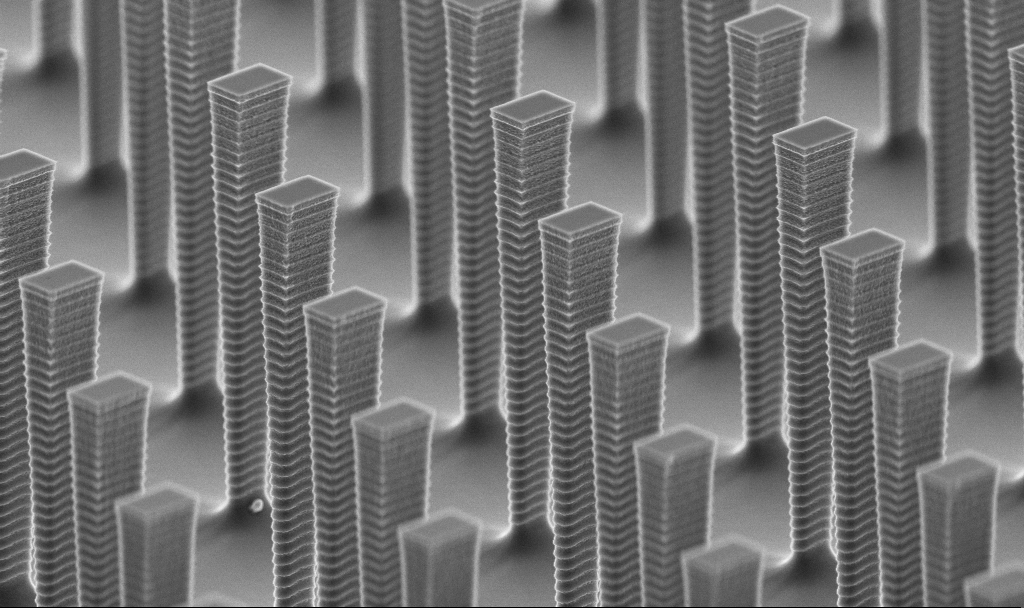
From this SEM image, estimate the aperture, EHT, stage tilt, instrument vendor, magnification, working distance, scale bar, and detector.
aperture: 30 µm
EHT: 5 kV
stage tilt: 70°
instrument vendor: Zeiss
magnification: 9.9 K X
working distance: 6.2 mm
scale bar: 2000 nm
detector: InLens